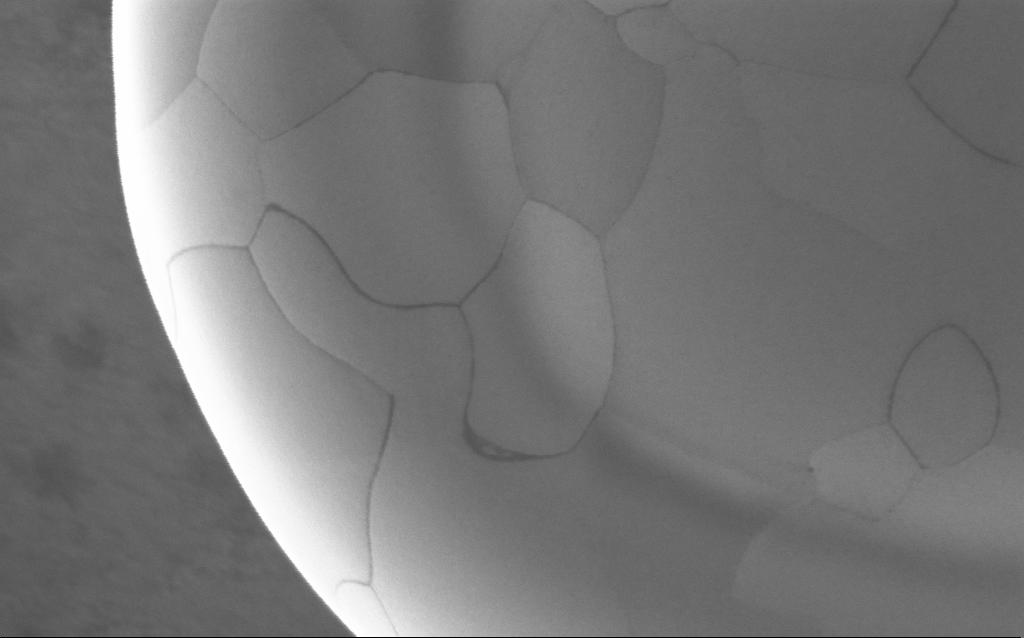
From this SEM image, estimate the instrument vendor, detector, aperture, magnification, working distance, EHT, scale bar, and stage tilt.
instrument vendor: Zeiss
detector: InLens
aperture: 30 µm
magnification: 152.95 K X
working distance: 2 mm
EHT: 5 kV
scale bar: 200 nm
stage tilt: -0°